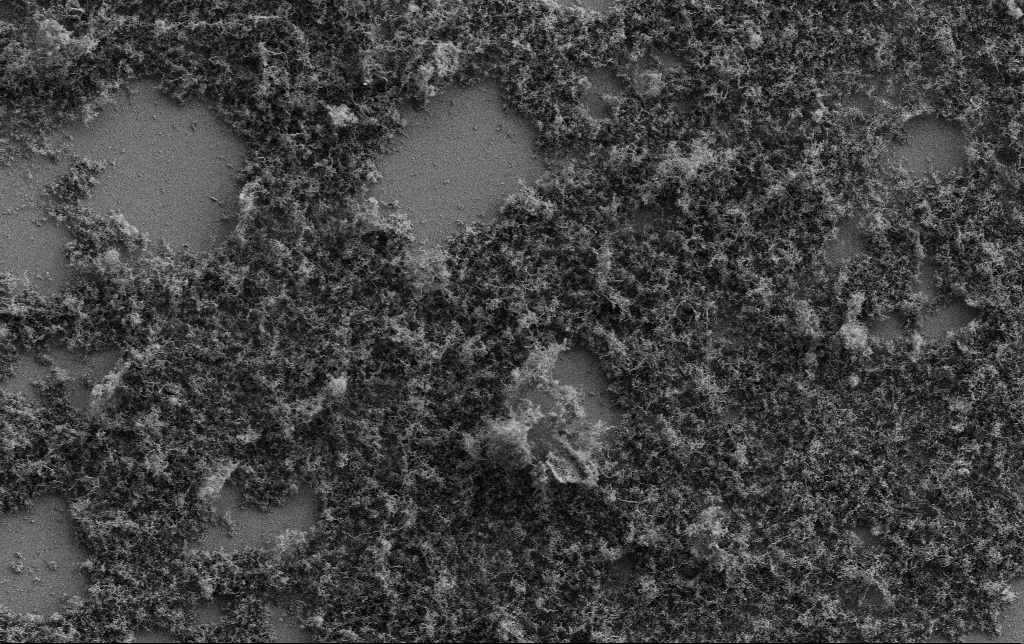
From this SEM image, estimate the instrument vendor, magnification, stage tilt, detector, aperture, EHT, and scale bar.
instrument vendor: Zeiss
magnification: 2.5 K X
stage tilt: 0°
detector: SE2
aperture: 30 µm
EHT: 2 kV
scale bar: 10000 nm